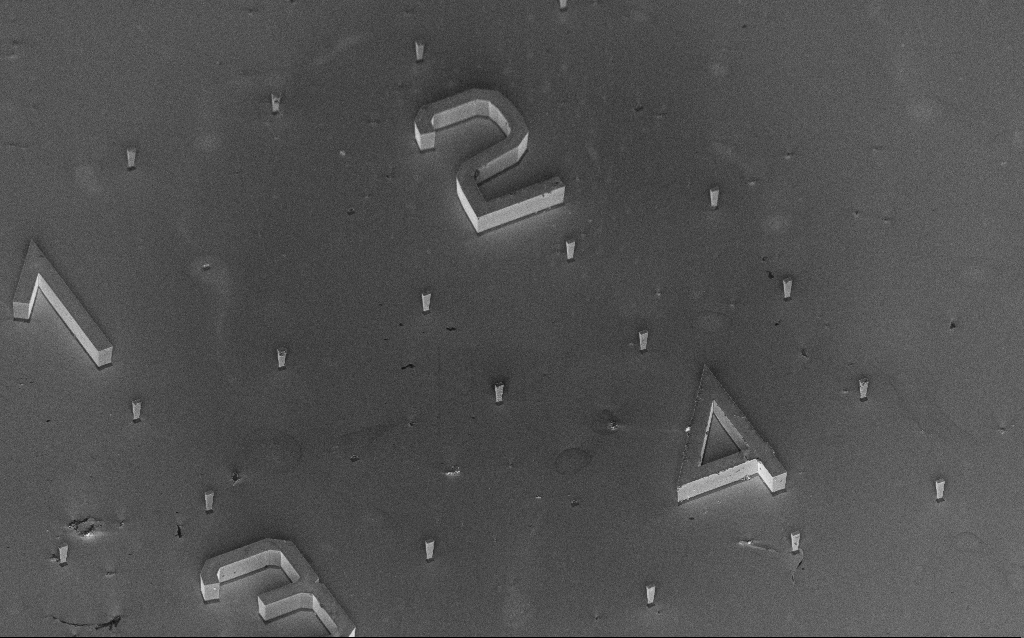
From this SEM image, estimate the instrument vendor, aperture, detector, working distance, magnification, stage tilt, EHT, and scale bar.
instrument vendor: Zeiss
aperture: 30 µm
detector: InLens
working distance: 12 mm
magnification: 0.596 K X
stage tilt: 45°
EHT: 5 kV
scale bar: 100000 nm